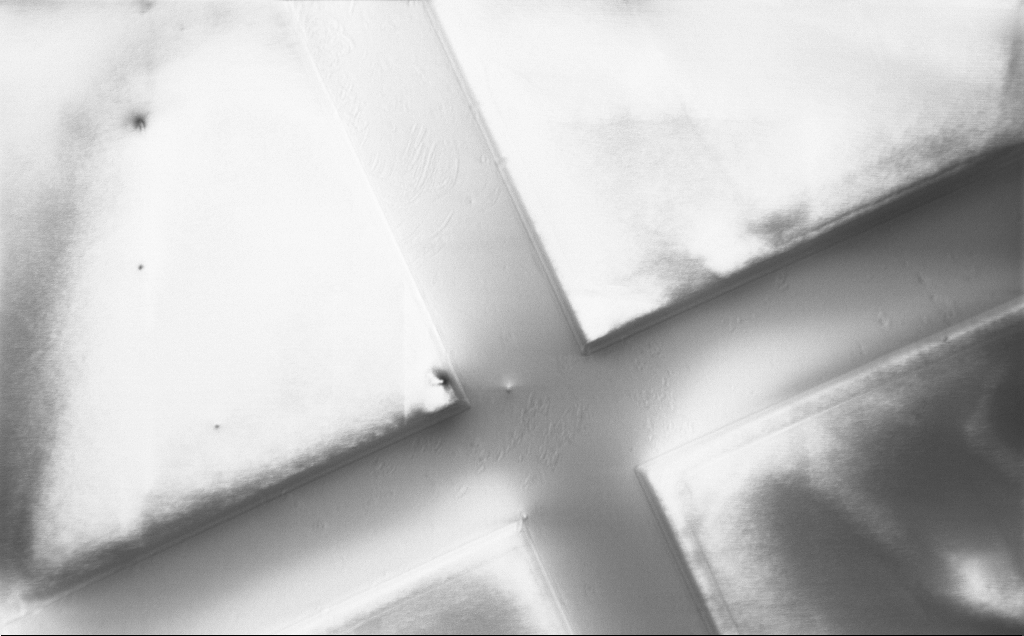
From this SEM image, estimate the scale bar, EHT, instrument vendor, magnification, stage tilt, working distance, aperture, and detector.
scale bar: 100000 nm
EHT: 1 kV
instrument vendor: Zeiss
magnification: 0.455 K X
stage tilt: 0°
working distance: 5 mm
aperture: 30 µm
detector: InLens